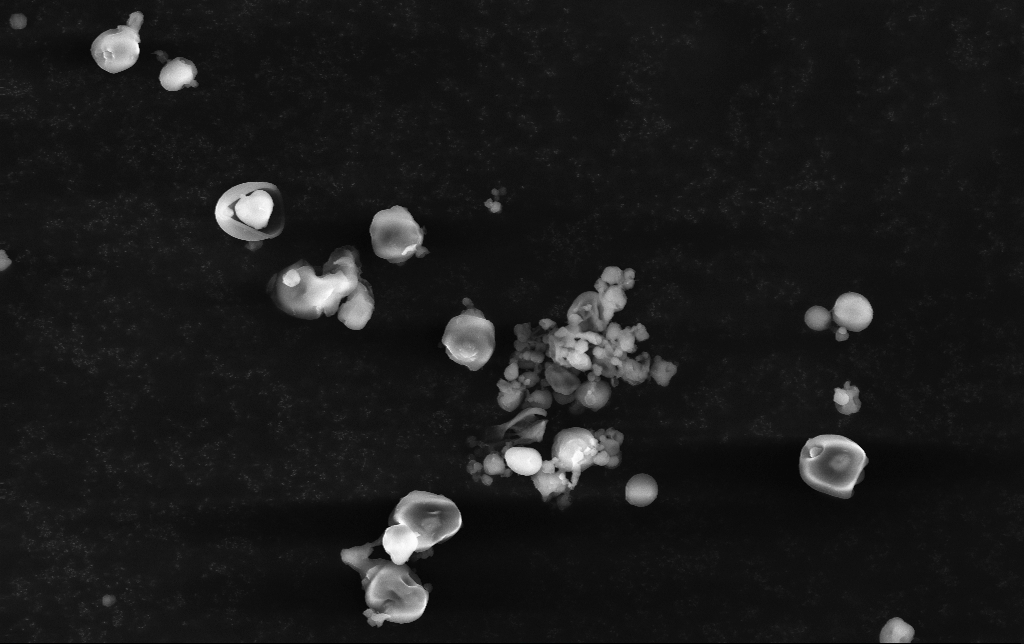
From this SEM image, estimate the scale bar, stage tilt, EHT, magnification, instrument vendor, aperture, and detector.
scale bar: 10000 nm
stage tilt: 0°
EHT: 15 kV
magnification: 4.1 K X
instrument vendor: Zeiss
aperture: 30 µm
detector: InLens